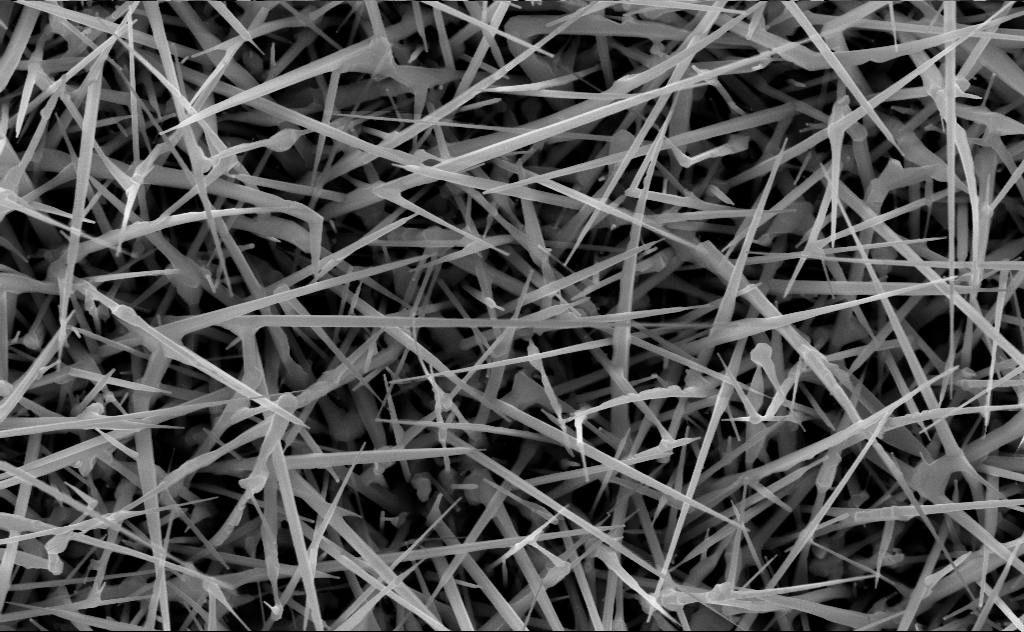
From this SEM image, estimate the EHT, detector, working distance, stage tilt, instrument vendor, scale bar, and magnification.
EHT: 10 kV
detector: InLens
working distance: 10 mm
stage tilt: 0°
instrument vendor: Zeiss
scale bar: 1000 nm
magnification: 40 K X